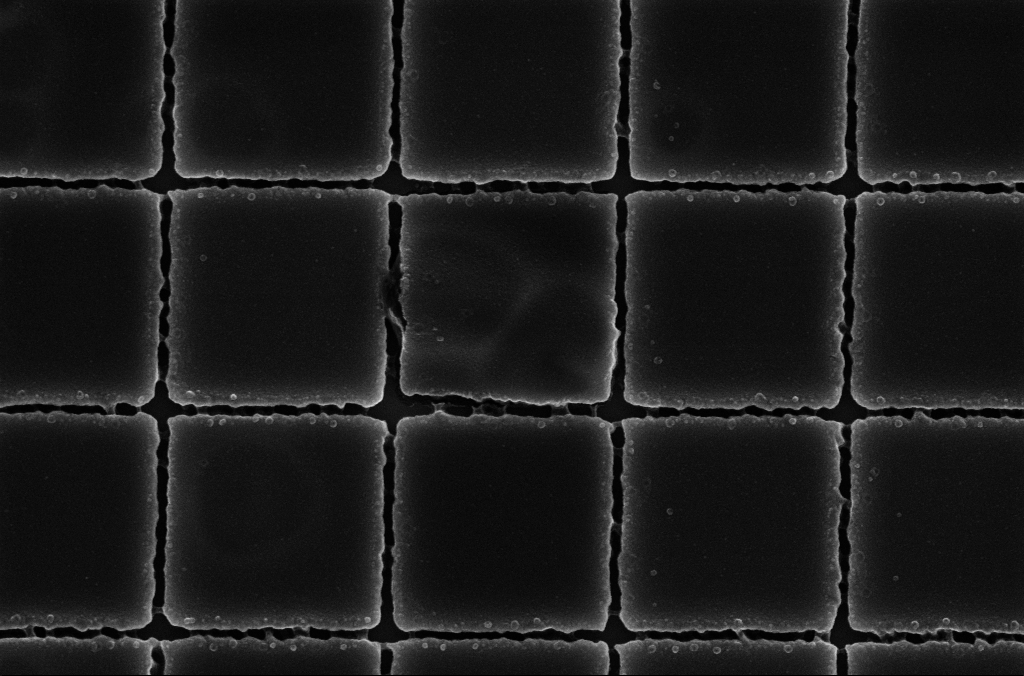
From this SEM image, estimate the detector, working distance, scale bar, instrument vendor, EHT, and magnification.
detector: InLens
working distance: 3.9 mm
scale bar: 1000 nm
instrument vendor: Zeiss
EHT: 5 kV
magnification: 42.4 K X